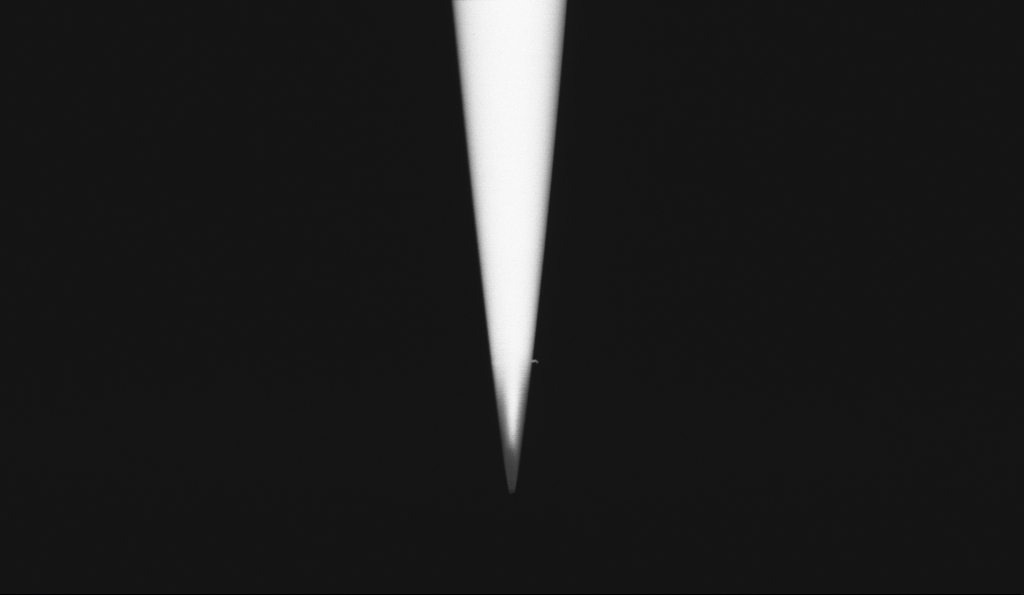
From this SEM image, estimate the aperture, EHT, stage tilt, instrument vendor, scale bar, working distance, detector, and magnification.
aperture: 30 µm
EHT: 1 kV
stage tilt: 0°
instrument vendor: Zeiss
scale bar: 2000 nm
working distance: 5.6 mm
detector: InLens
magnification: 25 K X